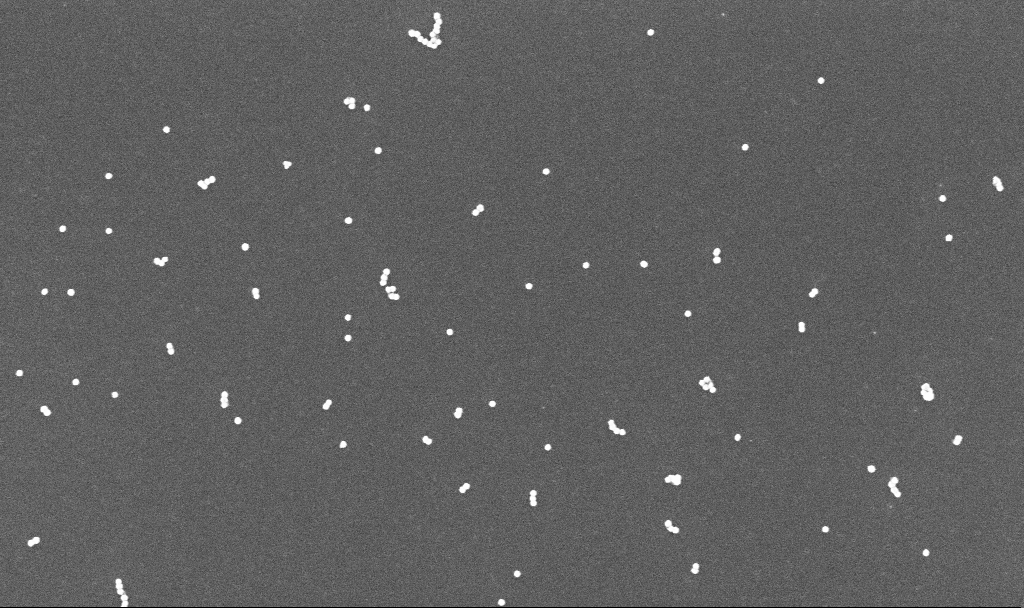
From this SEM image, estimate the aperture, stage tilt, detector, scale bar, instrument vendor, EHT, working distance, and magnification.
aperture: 30 µm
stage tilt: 0°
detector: InLens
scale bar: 200 nm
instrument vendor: Zeiss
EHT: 10 kV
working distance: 3.4 mm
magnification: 100 K X